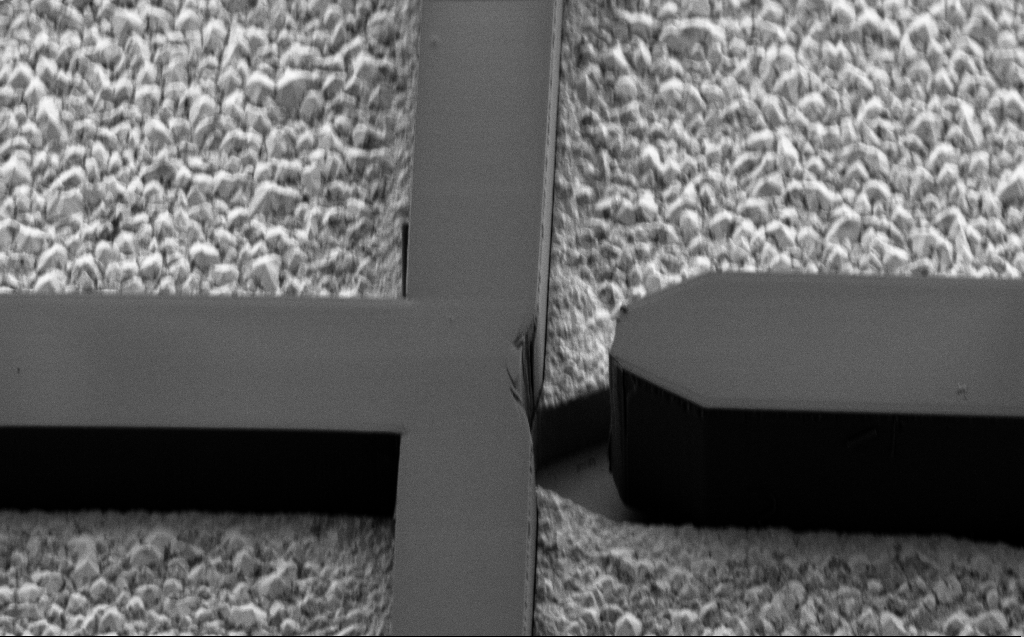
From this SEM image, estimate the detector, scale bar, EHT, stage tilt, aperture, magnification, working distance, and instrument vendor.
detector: SE2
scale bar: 10000 nm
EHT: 1 kV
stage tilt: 45°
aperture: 30 µm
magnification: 4.07 K X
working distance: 7 mm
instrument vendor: Zeiss